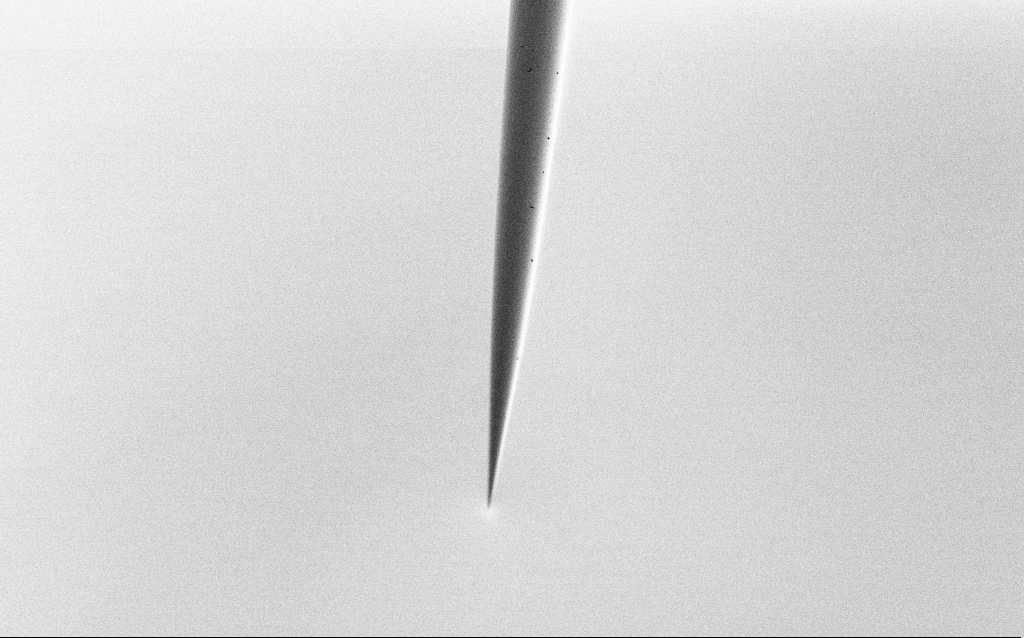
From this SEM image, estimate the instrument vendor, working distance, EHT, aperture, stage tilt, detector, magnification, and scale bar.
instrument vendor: Zeiss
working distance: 6 mm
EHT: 2 kV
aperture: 30 µm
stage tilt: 45°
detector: SE2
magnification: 1 K X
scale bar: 20000 nm